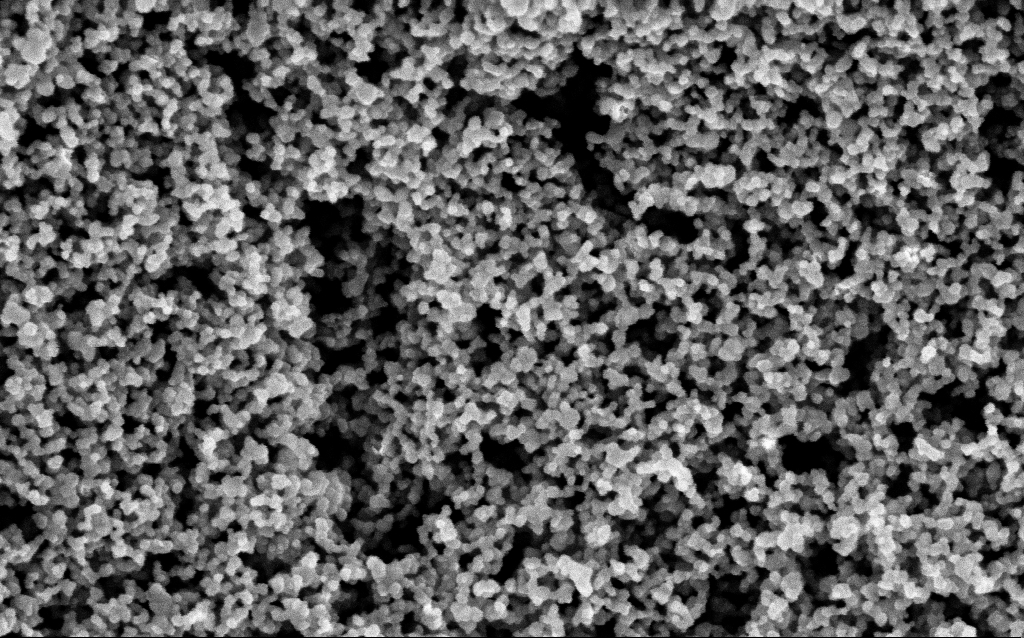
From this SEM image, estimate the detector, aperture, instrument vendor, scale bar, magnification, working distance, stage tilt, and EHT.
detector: InLens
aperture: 30 µm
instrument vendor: Zeiss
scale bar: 100 nm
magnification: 130 K X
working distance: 7.5 mm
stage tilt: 0°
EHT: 3 kV